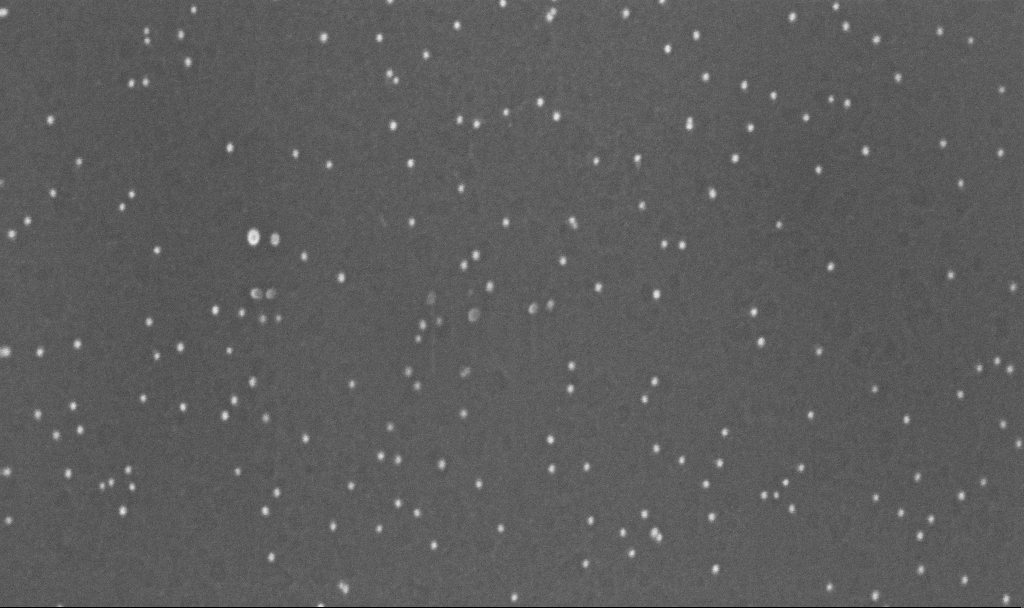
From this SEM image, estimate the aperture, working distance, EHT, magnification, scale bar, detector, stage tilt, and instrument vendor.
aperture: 30 µm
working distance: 3.2 mm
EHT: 10 kV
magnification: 200 K X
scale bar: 100 nm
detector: InLens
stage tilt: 0°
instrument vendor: Zeiss